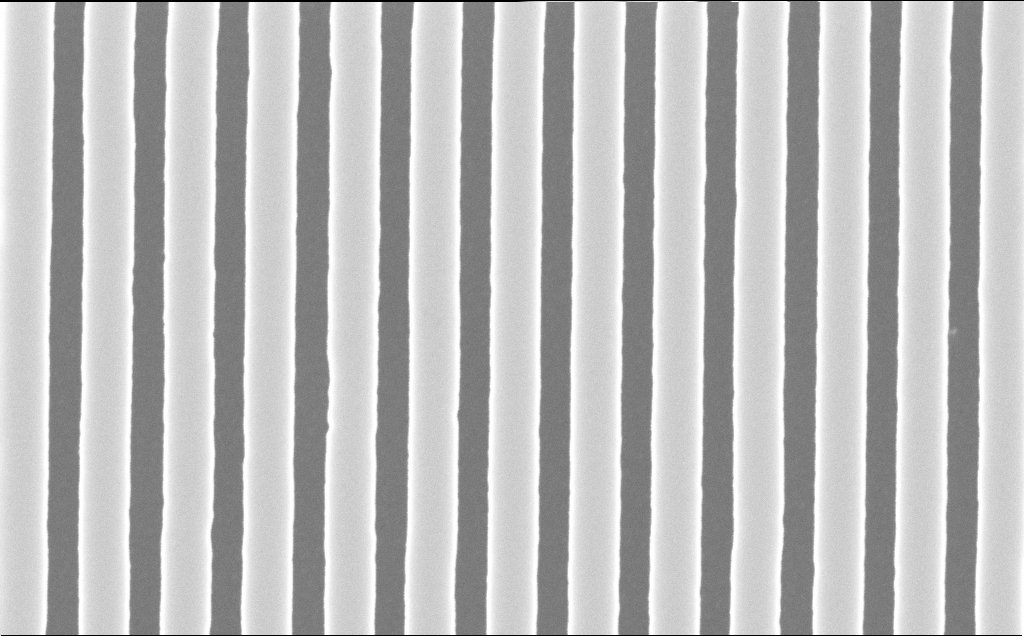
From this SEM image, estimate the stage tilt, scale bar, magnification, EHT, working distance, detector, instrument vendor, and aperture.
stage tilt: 0°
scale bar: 200 nm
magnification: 75 K X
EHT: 10 kV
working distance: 6 mm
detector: InLens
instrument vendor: Zeiss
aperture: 30 µm